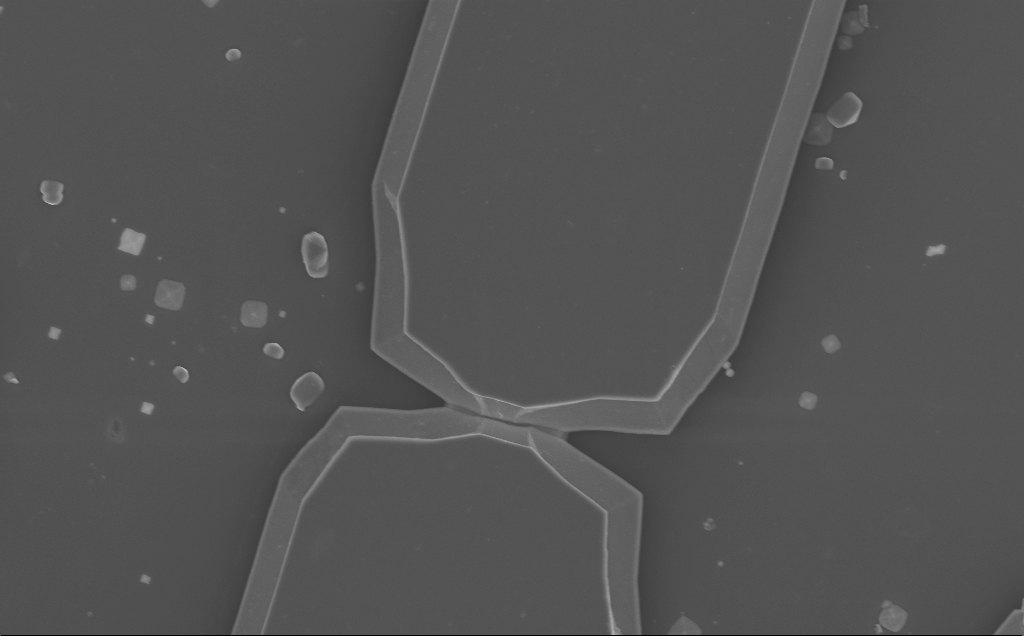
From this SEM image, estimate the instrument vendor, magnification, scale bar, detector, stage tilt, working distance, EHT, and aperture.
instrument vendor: Zeiss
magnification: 6.43 K X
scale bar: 10000 nm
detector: InLens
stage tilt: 0°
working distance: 10 mm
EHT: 5 kV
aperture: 30 µm